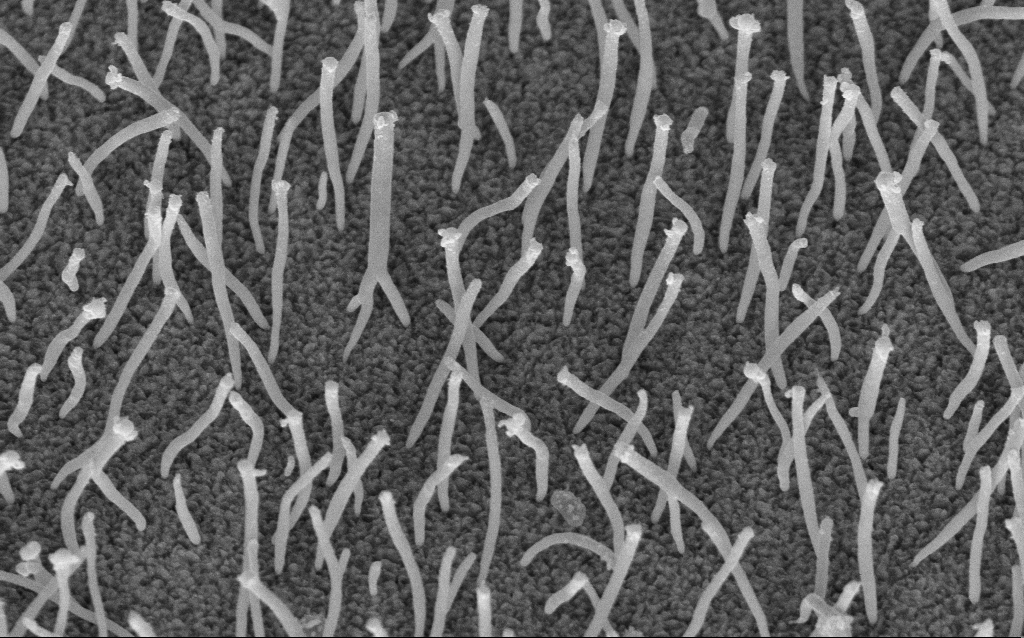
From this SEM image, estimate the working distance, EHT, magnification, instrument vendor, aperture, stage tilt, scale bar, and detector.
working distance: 8.3 mm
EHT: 5 kV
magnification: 50 K X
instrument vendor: Zeiss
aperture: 30 µm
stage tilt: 45°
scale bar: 1000 nm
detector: InLens